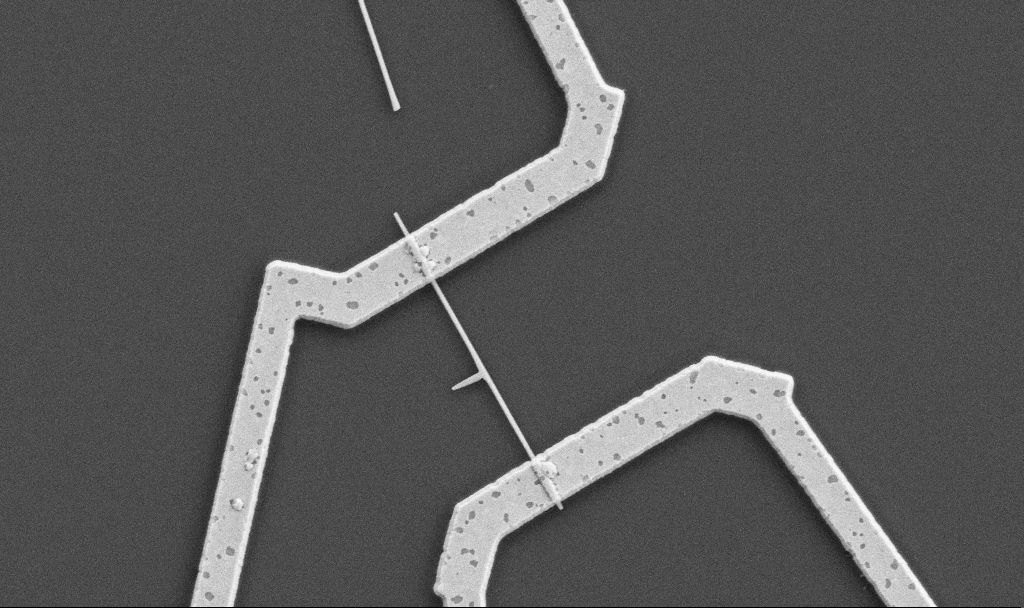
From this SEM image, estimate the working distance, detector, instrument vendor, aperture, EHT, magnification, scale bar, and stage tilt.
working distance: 10.7 mm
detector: SE2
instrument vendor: Zeiss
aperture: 30 µm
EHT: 5 kV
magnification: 20 K X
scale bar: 1000 nm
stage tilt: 0°